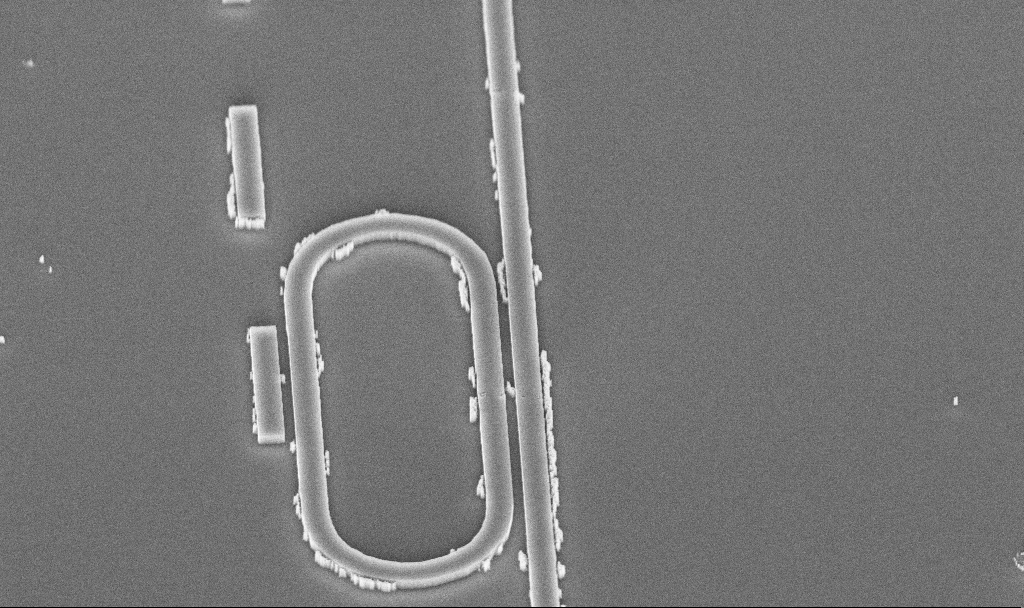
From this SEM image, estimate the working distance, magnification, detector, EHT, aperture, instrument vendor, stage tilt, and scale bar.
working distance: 10 mm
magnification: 19.43 K X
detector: InLens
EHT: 5 kV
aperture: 30 µm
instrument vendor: Zeiss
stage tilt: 45°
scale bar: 1000 nm